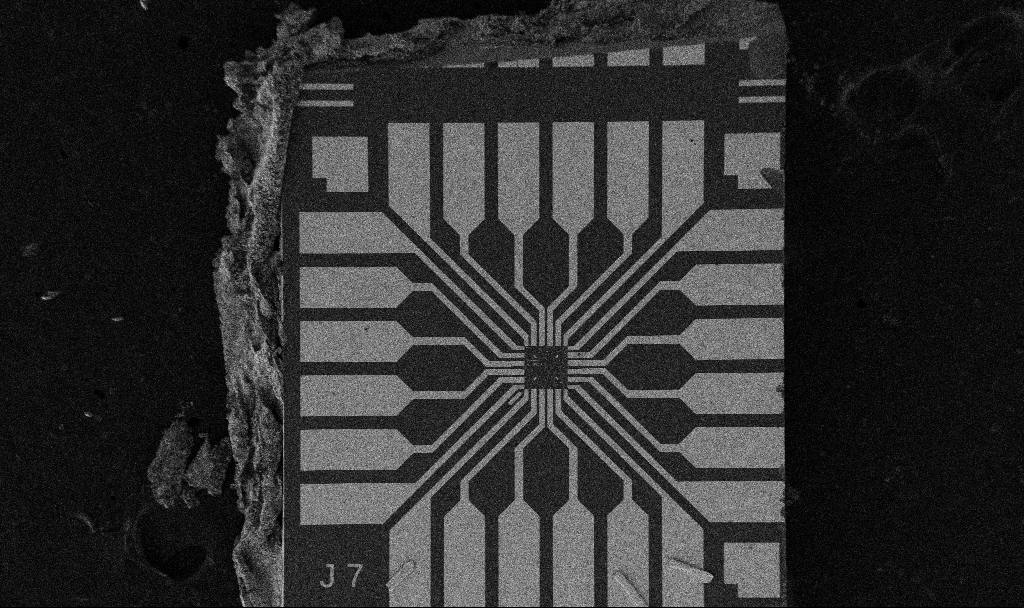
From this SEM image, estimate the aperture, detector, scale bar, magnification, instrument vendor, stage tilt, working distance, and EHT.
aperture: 30 µm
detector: SE2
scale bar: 200000 nm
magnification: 0.1 K X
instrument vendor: Zeiss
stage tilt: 0°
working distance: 10.7 mm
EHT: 5 kV